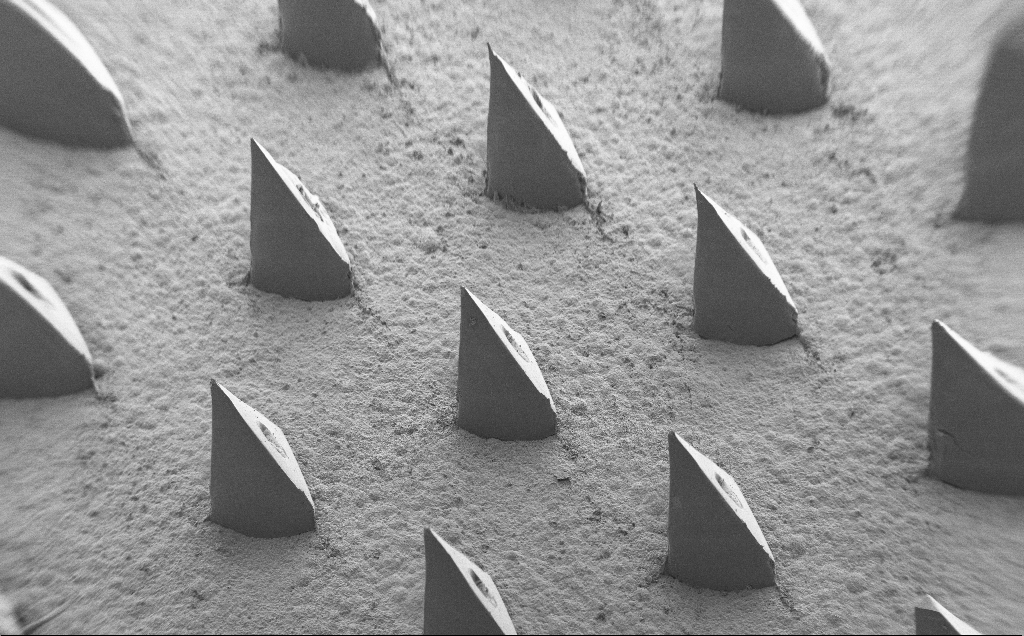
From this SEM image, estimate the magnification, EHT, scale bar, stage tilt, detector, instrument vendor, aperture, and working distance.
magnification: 0.067 K X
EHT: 5 kV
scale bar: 1e+06 nm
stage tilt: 40°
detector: SE2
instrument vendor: Zeiss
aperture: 30 µm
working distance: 9 mm